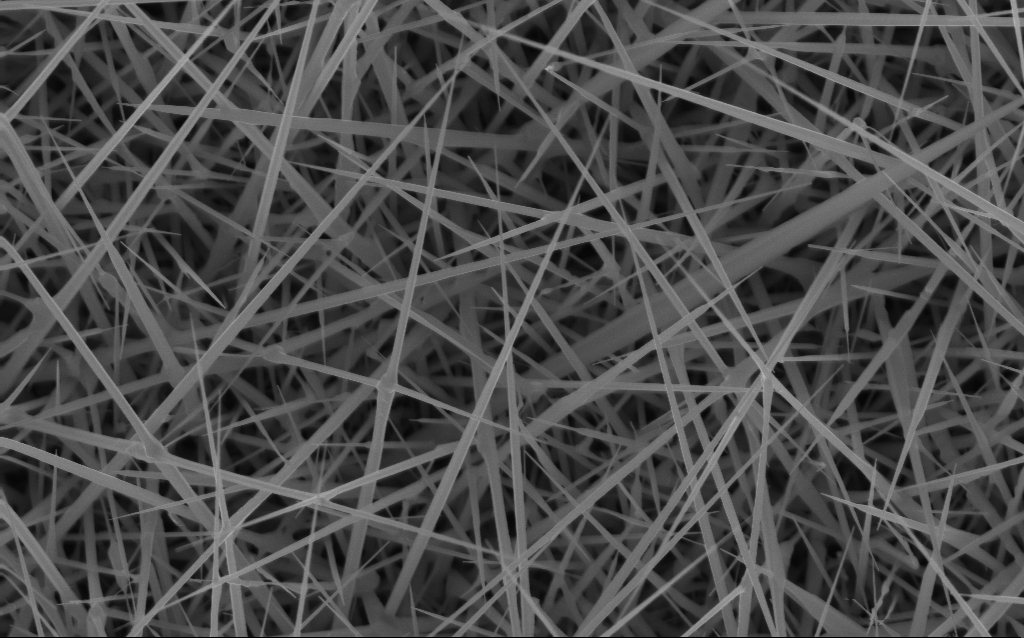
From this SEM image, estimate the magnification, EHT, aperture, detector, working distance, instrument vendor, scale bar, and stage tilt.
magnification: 20 K X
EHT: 10 kV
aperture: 30 µm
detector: InLens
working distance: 4 mm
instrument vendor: Zeiss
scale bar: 1000 nm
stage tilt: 0°